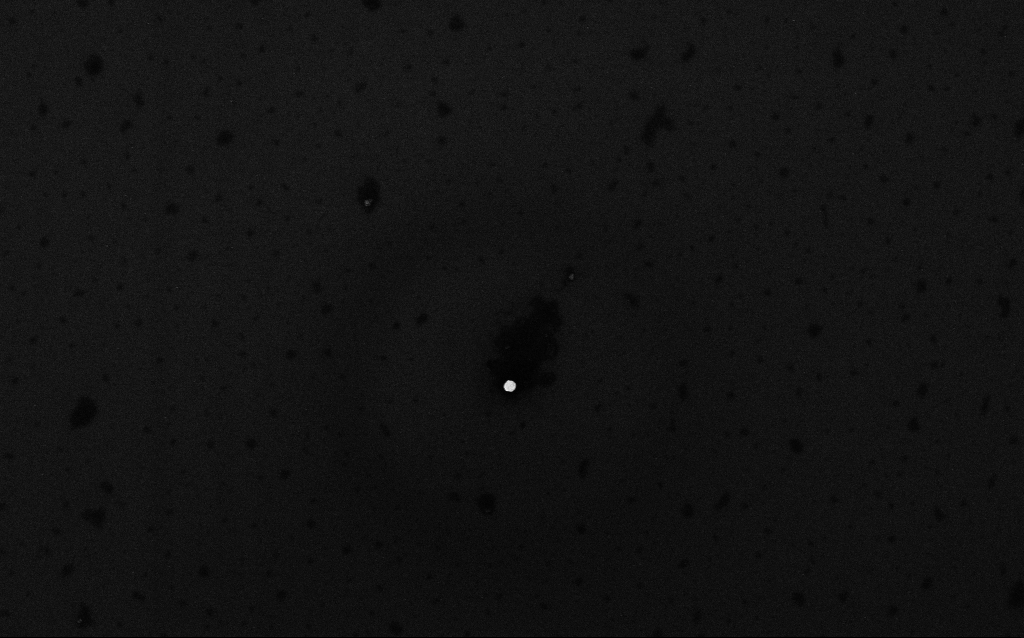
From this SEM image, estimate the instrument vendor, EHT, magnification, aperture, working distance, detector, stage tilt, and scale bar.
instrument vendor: Zeiss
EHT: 10 kV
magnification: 67.84 K X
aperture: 30 µm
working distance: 3.1 mm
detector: InLens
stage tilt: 0°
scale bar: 1000 nm